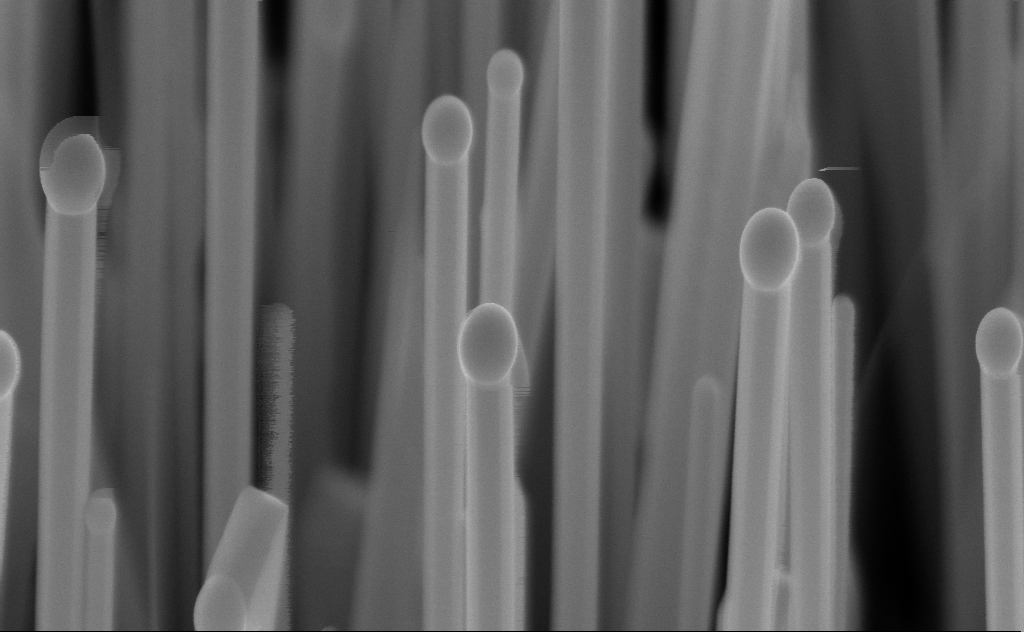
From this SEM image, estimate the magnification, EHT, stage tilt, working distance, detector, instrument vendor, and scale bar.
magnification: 144.2 K X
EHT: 10 kV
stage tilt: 45°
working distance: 6 mm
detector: InLens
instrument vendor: Zeiss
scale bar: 200 nm